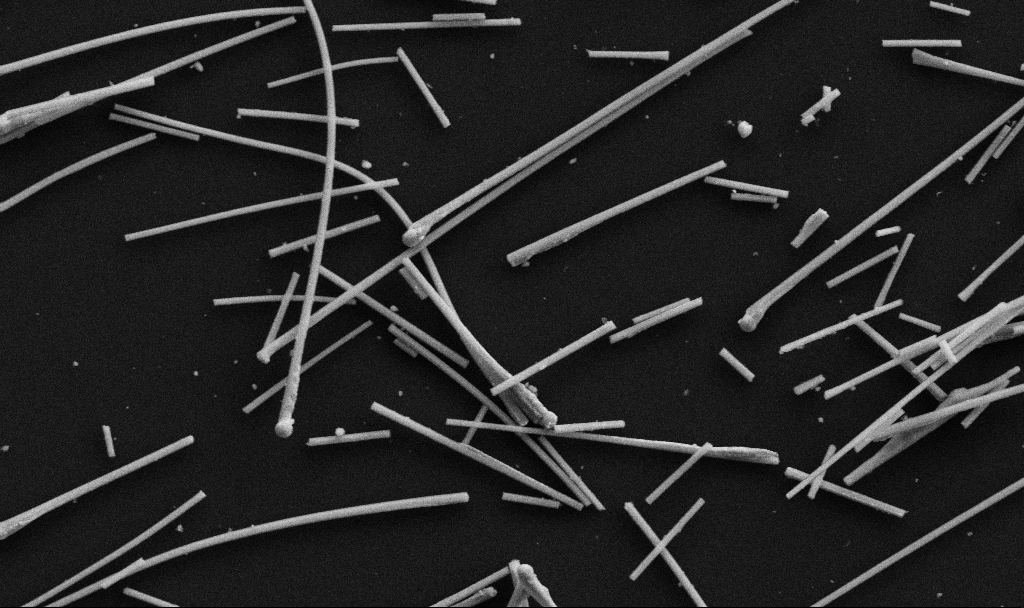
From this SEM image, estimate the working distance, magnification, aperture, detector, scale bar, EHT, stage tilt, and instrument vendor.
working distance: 6.7 mm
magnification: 29.49 K X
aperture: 30 µm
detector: SE2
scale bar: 1000 nm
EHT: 5 kV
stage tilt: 0°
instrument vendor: Zeiss